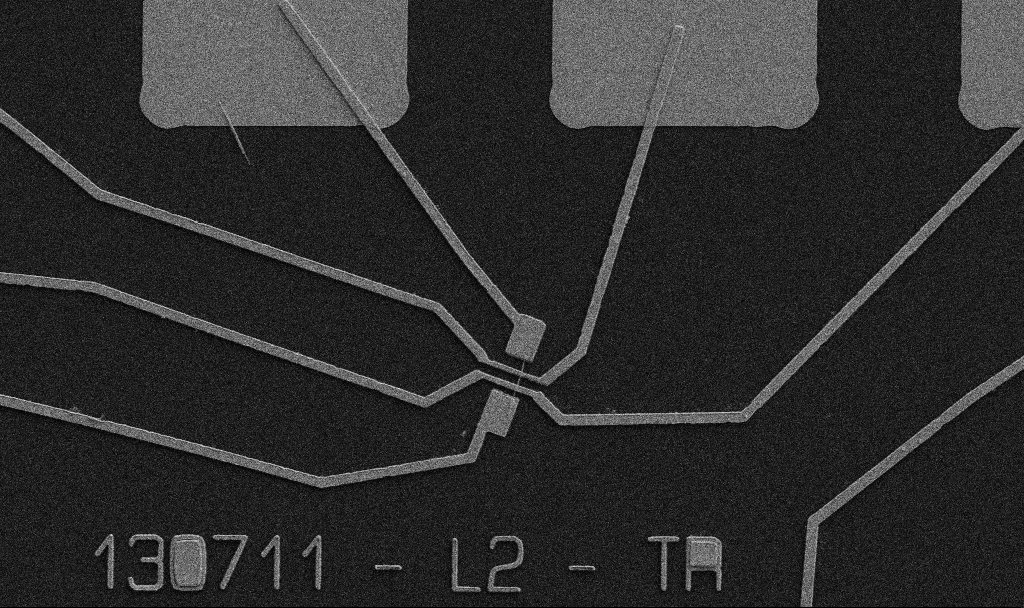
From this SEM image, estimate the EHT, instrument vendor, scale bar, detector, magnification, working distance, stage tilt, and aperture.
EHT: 5 kV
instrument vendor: Zeiss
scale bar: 10000 nm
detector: SE2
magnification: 5 K X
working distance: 10.7 mm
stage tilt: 0°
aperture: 30 µm